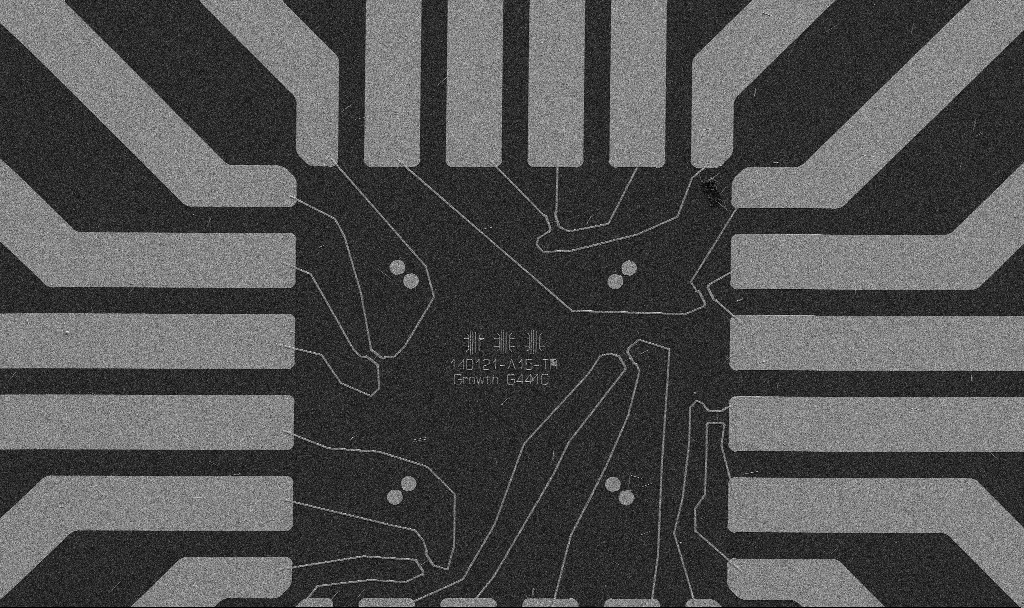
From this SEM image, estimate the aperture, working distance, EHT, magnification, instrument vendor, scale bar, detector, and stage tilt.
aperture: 30 µm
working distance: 10.7 mm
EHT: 5 kV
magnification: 1 K X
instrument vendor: Zeiss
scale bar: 20000 nm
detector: SE2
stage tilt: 0°